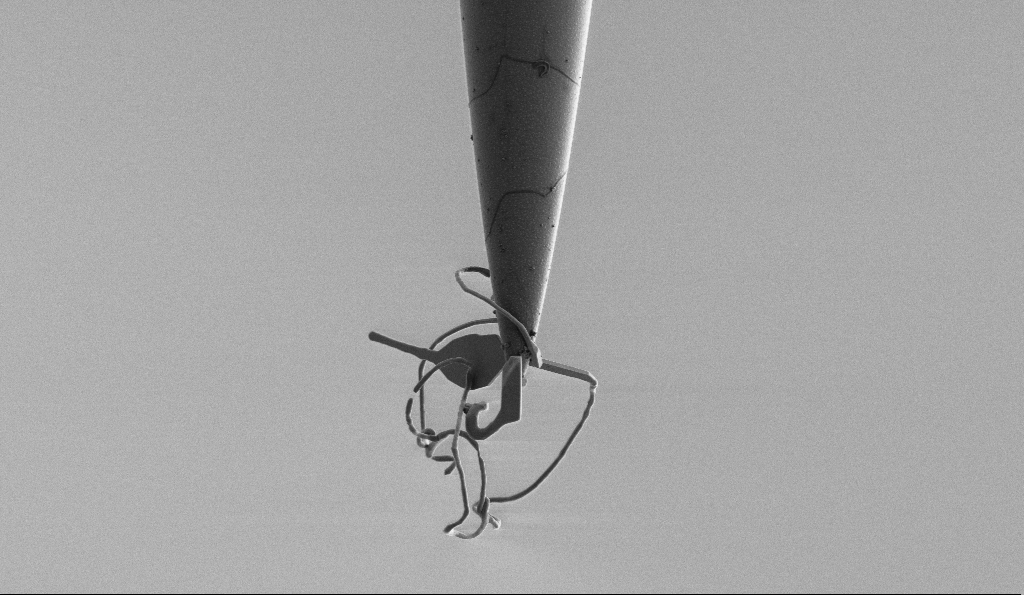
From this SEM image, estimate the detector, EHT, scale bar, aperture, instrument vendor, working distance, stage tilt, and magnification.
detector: SE2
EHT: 1 kV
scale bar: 10000 nm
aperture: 30 µm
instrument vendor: Zeiss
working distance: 6.6 mm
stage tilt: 0°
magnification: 5 K X